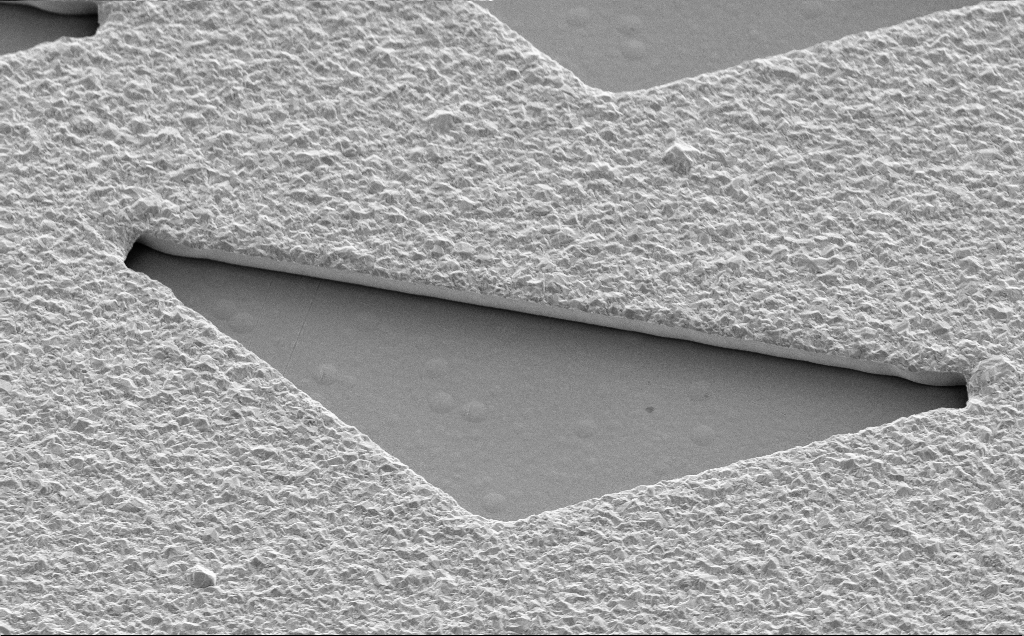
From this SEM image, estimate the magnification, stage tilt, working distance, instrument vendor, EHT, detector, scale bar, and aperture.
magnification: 8.16 K X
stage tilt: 35°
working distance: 13 mm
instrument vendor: Zeiss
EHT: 5 kV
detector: SE2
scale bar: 2000 nm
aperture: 30 µm